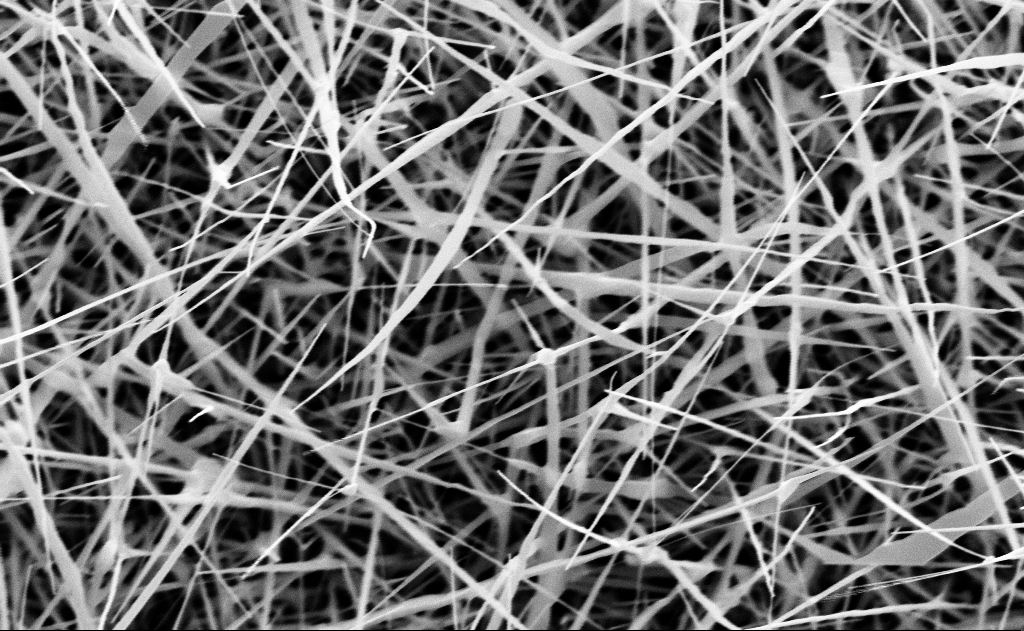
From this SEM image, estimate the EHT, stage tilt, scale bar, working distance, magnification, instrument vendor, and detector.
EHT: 10 kV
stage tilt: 0°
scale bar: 1000 nm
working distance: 15 mm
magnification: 40 K X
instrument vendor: Zeiss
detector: InLens